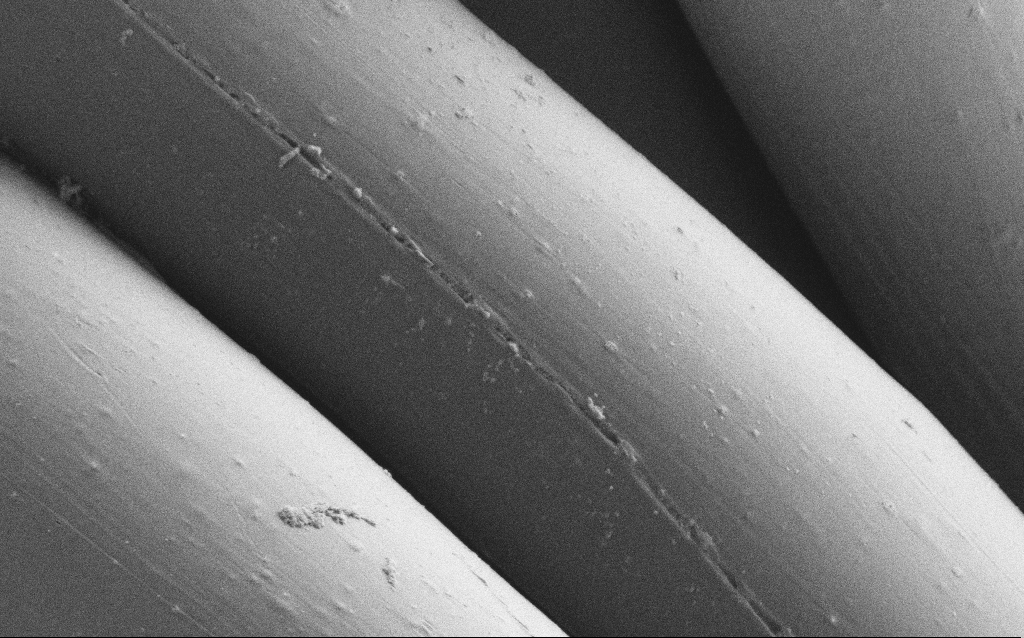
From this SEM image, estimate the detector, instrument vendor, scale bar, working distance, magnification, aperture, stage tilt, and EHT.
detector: SE2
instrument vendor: Zeiss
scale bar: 10000 nm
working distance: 4 mm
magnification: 6.42 K X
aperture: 30 µm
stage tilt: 0°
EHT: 1 kV